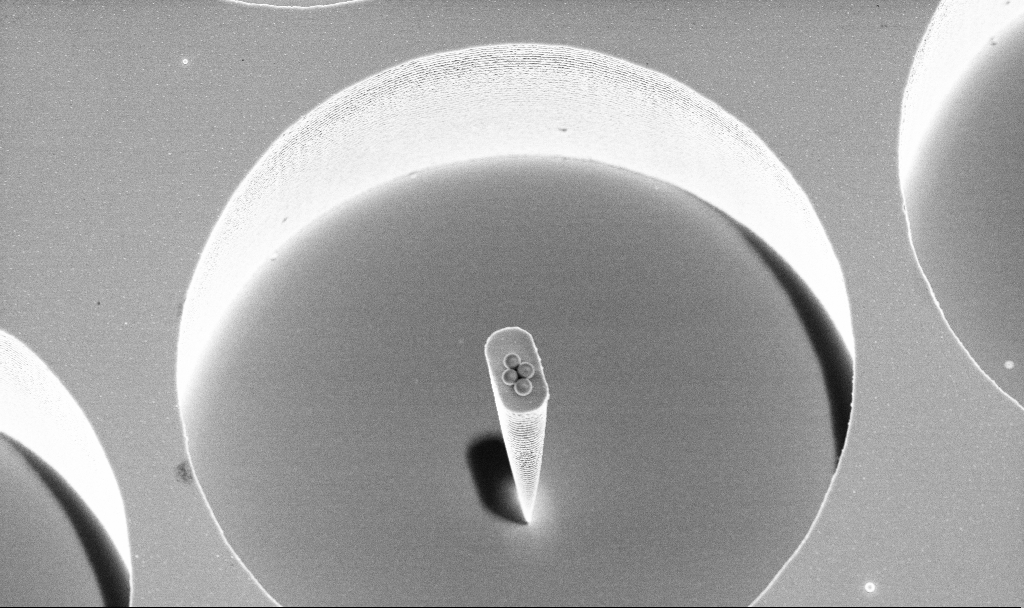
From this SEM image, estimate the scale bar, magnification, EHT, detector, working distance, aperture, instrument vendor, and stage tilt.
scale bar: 2000 nm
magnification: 8.39 K X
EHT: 4 kV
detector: InLens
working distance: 4.7 mm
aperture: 30 µm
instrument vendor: Zeiss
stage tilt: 15°